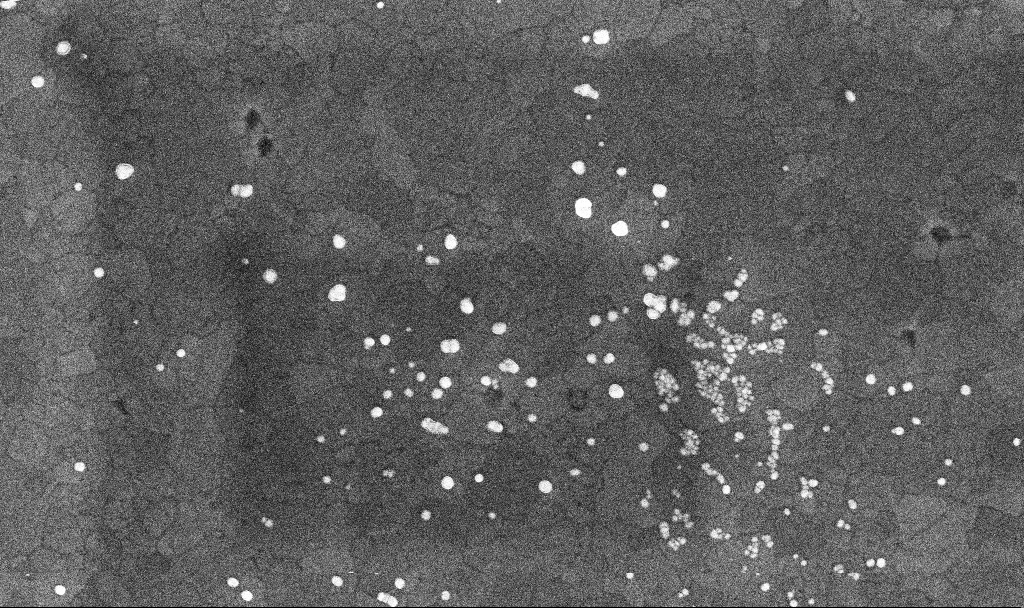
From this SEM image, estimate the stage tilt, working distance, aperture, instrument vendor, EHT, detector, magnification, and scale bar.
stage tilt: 0°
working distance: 3.4 mm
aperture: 30 µm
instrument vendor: Zeiss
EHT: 10 kV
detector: InLens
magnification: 78.04 K X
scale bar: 200 nm